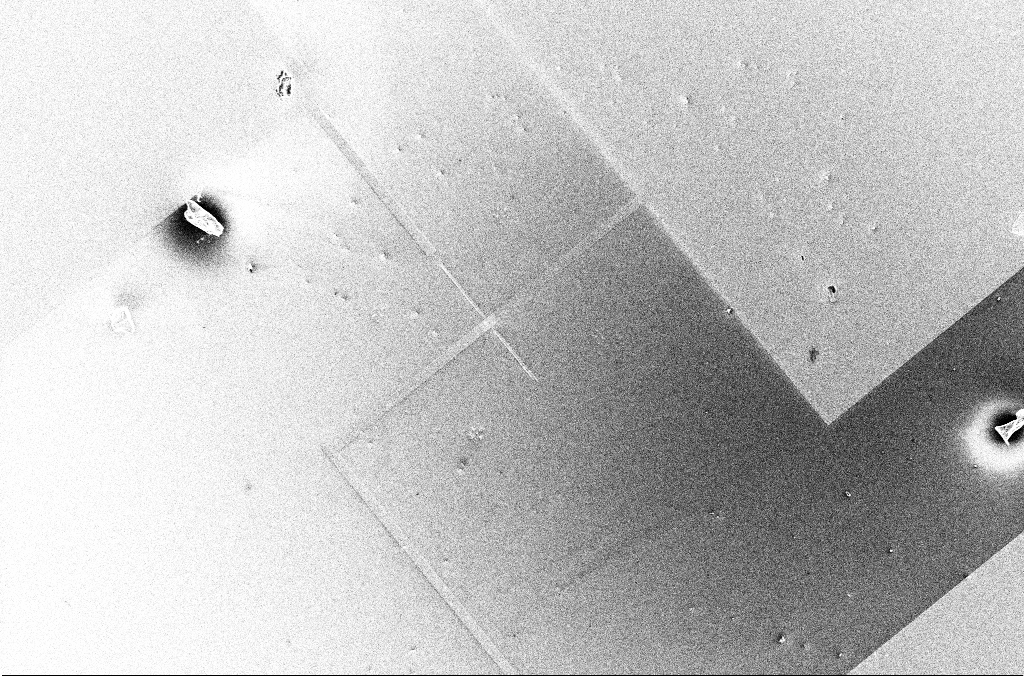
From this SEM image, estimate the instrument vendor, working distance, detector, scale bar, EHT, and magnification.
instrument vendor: Zeiss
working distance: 3.4 mm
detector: InLens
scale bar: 20000 nm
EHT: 5 kV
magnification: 0.729 K X